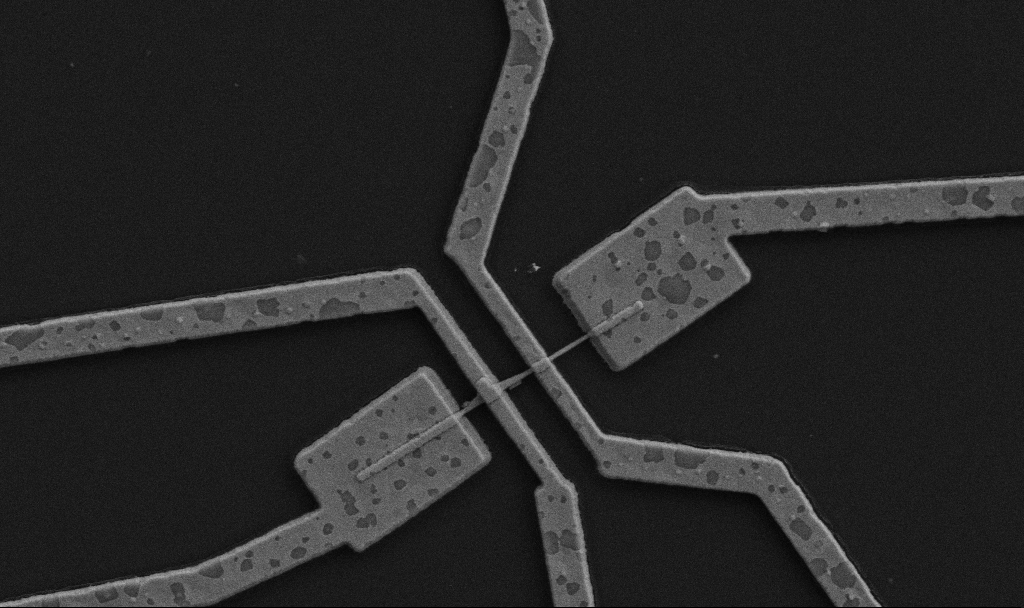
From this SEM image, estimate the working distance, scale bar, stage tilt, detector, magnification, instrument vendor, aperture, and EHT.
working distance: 10.7 mm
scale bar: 1000 nm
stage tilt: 0°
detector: SE2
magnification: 20 K X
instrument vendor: Zeiss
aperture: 30 µm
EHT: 5 kV